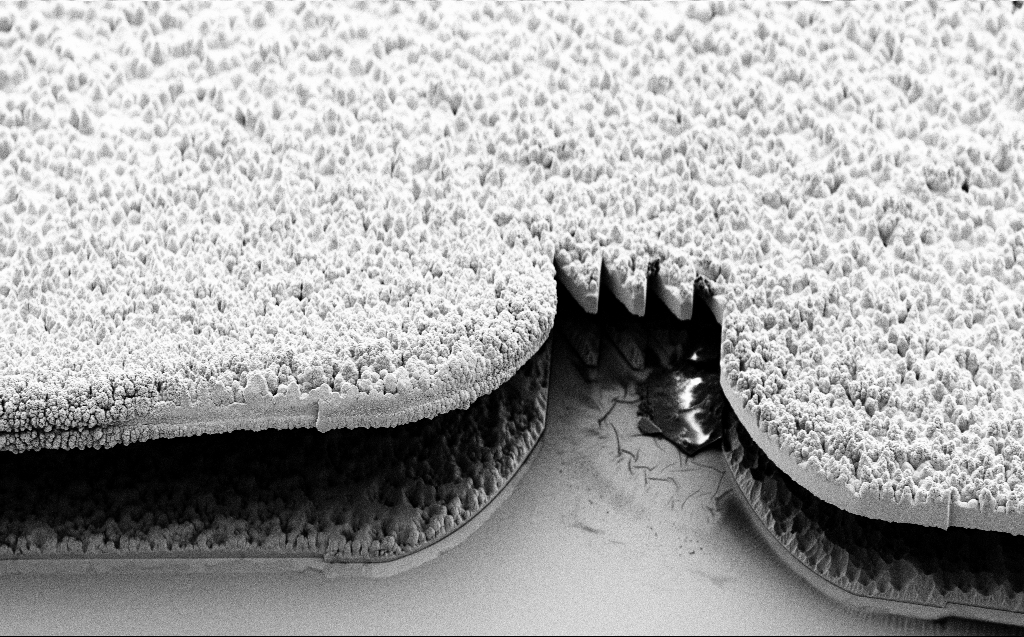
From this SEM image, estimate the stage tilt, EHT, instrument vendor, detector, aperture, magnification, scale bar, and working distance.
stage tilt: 45.1°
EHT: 5 kV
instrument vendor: Zeiss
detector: SE2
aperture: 30 µm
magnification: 1.54 K X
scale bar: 20000 nm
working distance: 9 mm